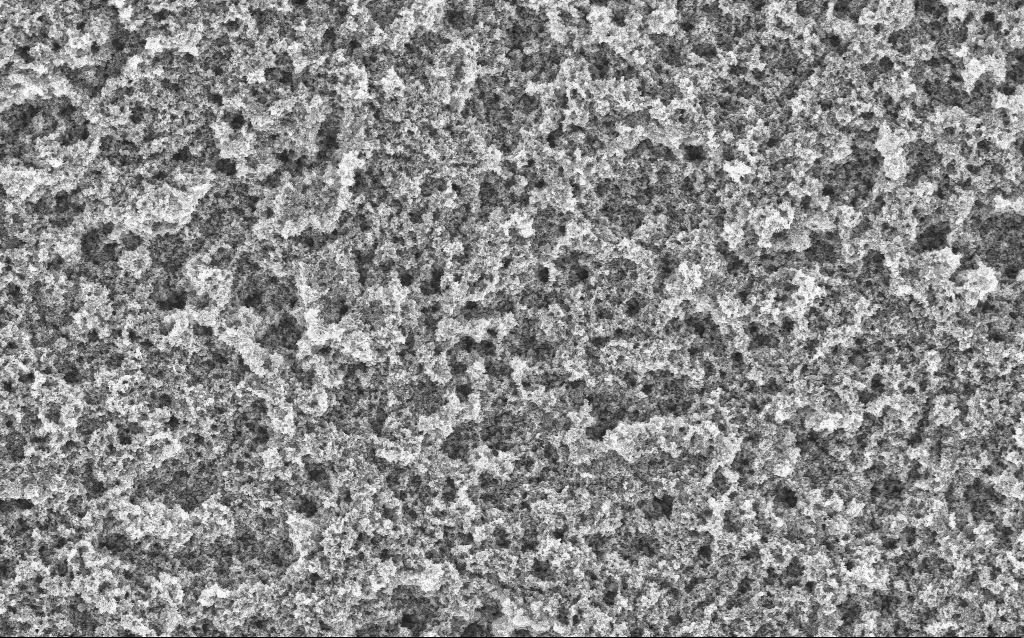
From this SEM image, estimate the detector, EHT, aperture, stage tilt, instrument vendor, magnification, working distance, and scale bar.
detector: InLens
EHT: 5 kV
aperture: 30 µm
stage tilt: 0°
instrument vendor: Zeiss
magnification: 20.87 K X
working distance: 4.4 mm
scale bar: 1000 nm